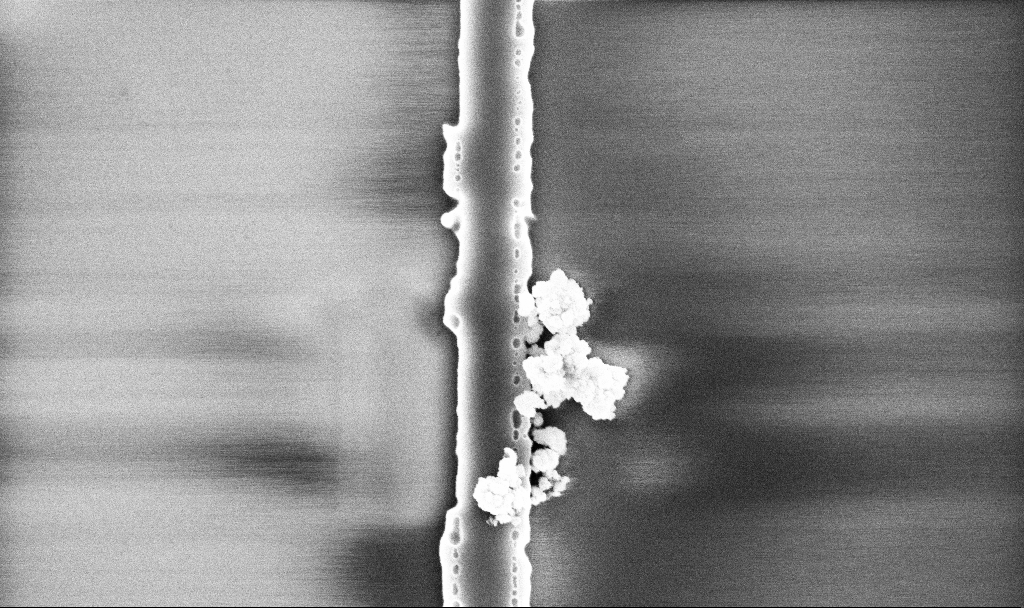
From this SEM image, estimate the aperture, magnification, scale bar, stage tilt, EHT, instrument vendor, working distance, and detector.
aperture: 30 µm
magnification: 40.2 K X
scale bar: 1000 nm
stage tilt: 0°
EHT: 5 kV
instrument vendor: Zeiss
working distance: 10.1 mm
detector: InLens